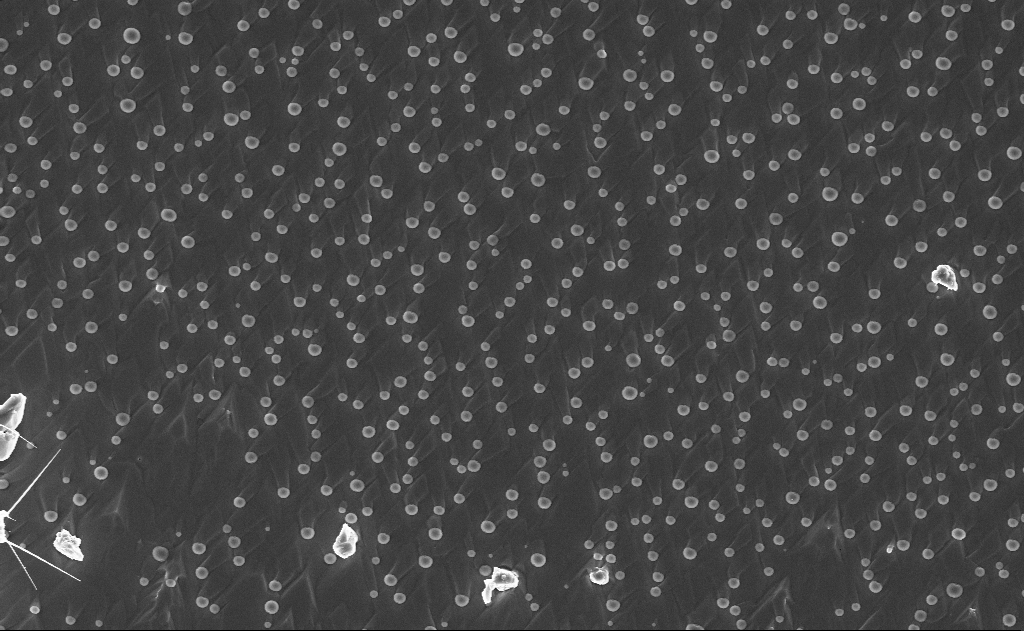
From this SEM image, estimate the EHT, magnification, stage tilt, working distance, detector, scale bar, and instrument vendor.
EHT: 10 kV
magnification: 5 K X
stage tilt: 0°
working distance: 10 mm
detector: InLens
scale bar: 10000 nm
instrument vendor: Zeiss